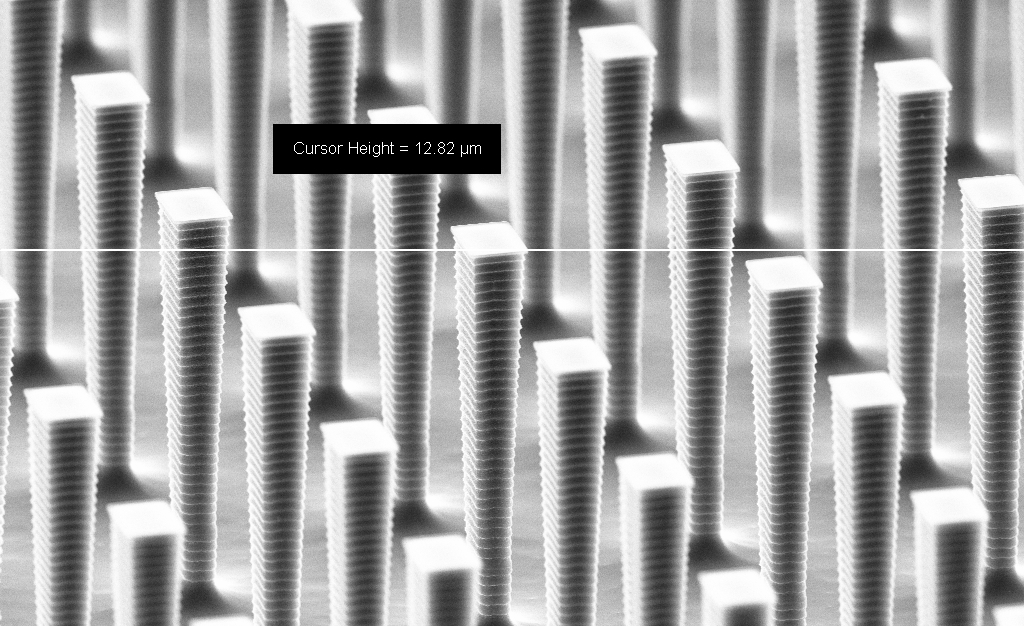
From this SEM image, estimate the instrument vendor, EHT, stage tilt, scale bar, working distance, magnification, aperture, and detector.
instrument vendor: Zeiss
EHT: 8 kV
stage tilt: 70°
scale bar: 2000 nm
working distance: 10.2 mm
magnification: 10.8 K X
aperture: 30 µm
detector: SE2